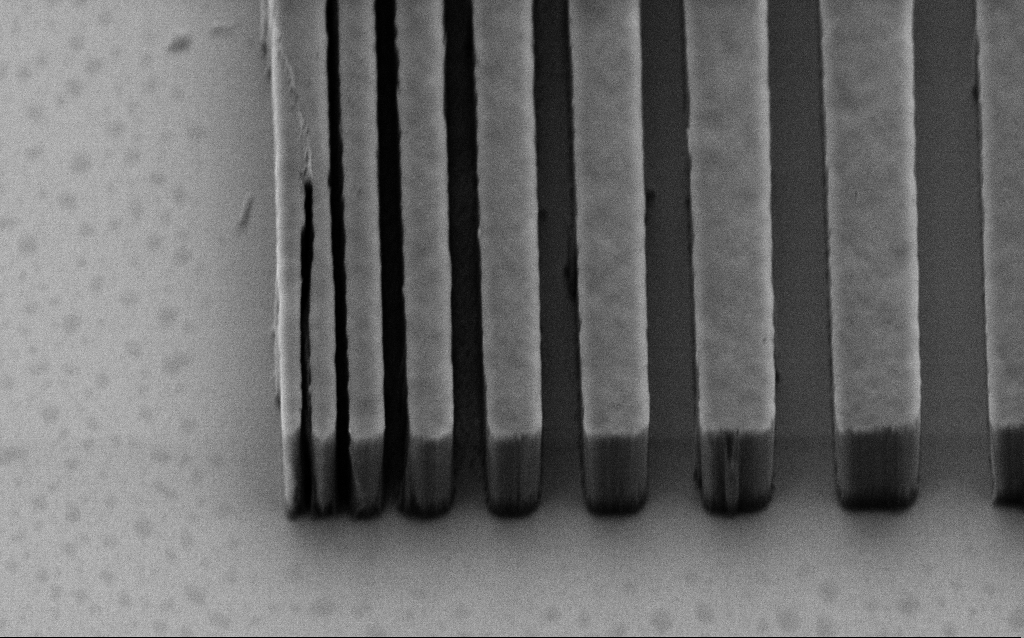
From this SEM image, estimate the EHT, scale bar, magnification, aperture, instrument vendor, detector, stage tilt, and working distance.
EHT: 3 kV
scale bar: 2000 nm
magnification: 35.23 K X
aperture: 30 µm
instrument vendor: Zeiss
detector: SE2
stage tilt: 45°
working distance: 9 mm